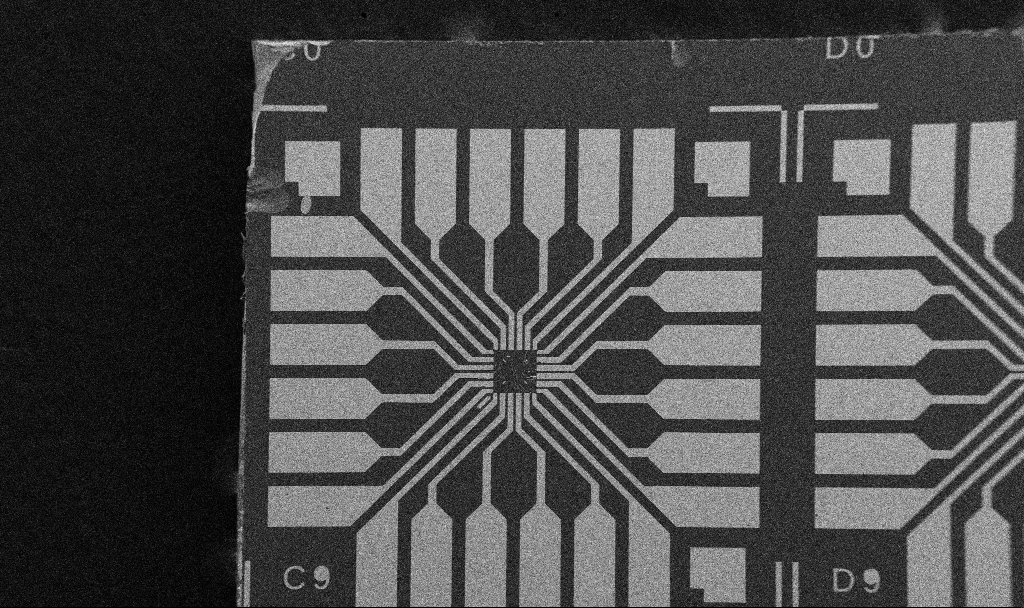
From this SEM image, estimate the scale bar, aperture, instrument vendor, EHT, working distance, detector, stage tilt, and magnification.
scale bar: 200000 nm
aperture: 30 µm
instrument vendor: Zeiss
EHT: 5 kV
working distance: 10.7 mm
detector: SE2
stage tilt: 0°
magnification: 0.1 K X